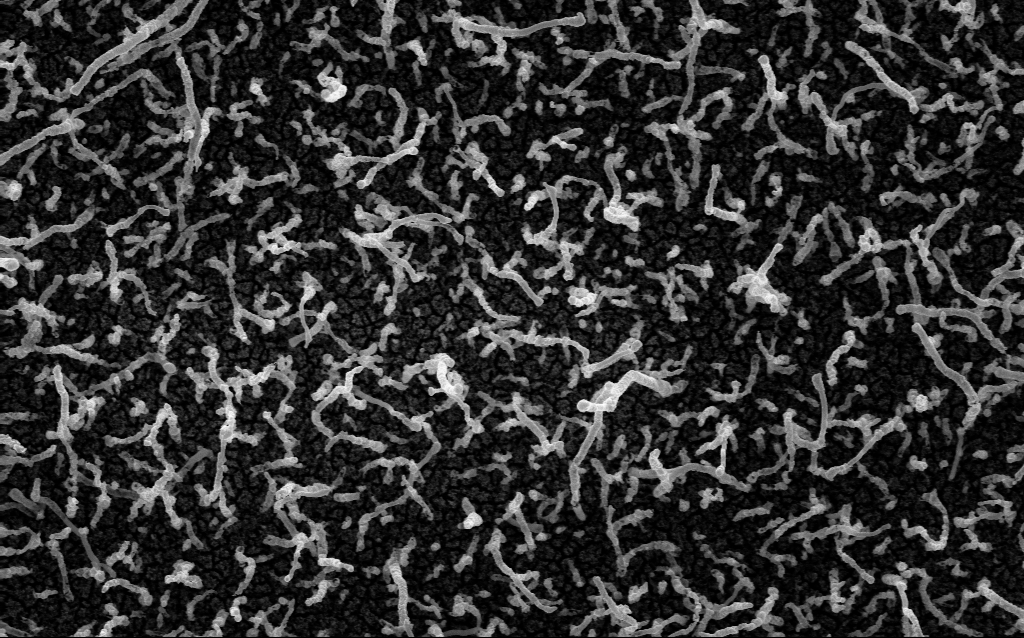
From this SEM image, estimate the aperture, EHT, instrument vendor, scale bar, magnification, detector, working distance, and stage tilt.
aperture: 30 µm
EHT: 5 kV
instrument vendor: Zeiss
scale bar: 1000 nm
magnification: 50 K X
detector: InLens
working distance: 2.1 mm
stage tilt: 0°